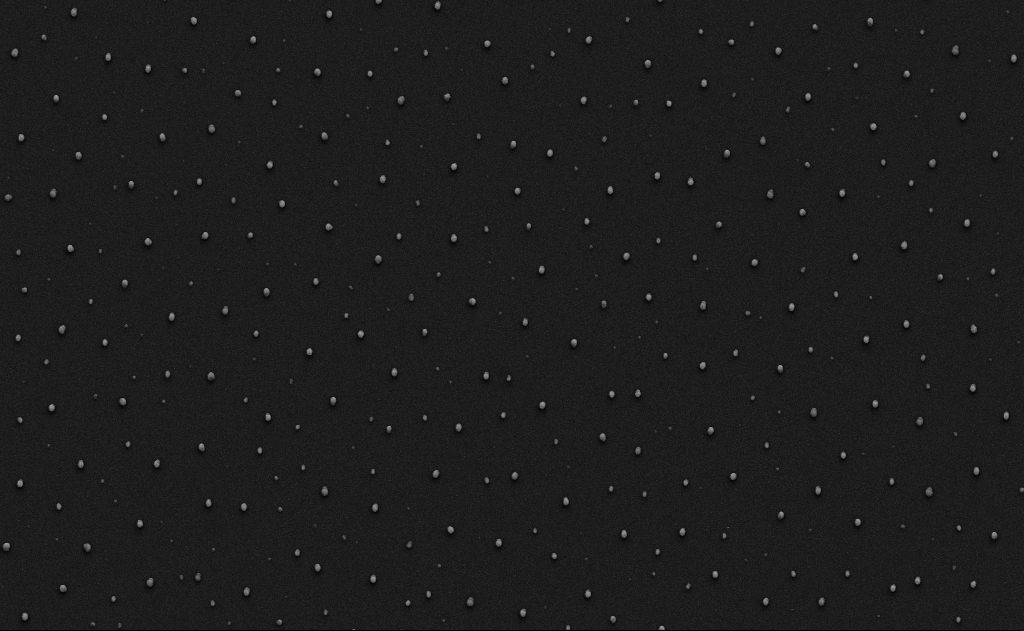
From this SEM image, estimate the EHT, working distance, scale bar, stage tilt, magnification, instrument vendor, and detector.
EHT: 10 kV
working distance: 9 mm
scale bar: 10000 nm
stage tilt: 0°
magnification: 5 K X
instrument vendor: Zeiss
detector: SE2